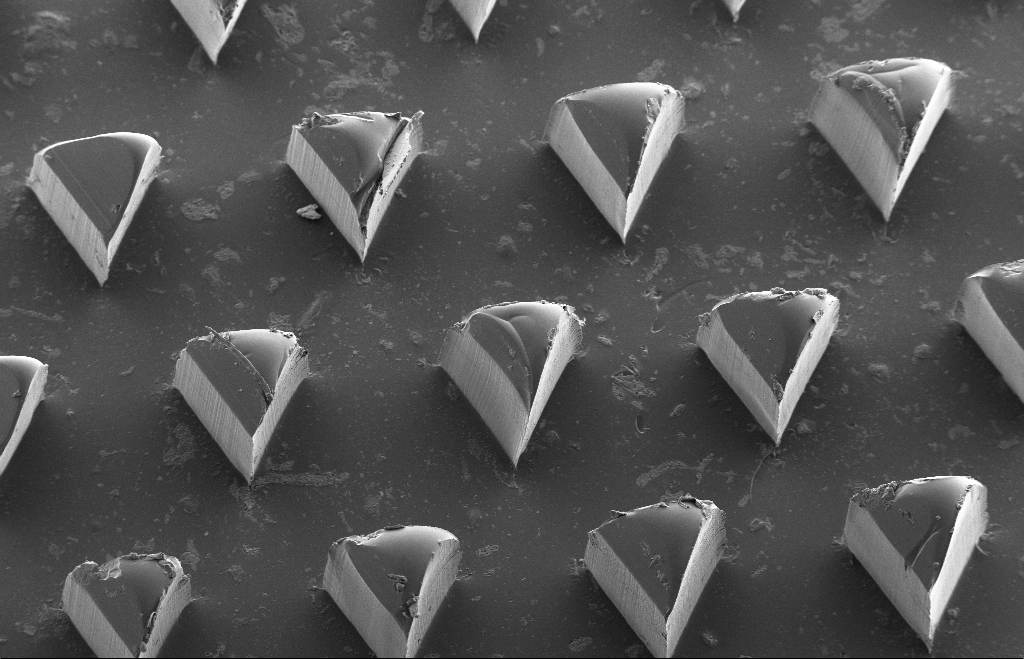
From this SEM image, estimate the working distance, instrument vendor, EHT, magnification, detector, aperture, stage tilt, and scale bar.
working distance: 13 mm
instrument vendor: Zeiss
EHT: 5 kV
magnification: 0.207 K X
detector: SE2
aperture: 30 µm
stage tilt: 22.5°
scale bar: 100000 nm